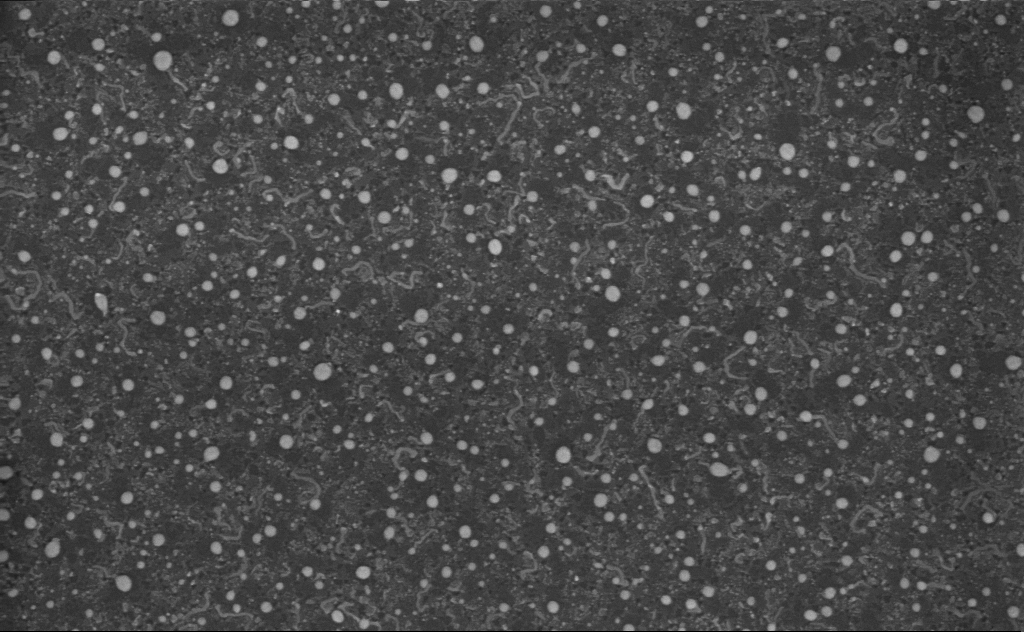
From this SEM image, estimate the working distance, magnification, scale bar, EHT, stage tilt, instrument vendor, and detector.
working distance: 4 mm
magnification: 80 K X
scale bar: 200 nm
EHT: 3 kV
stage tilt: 0°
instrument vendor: Zeiss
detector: InLens